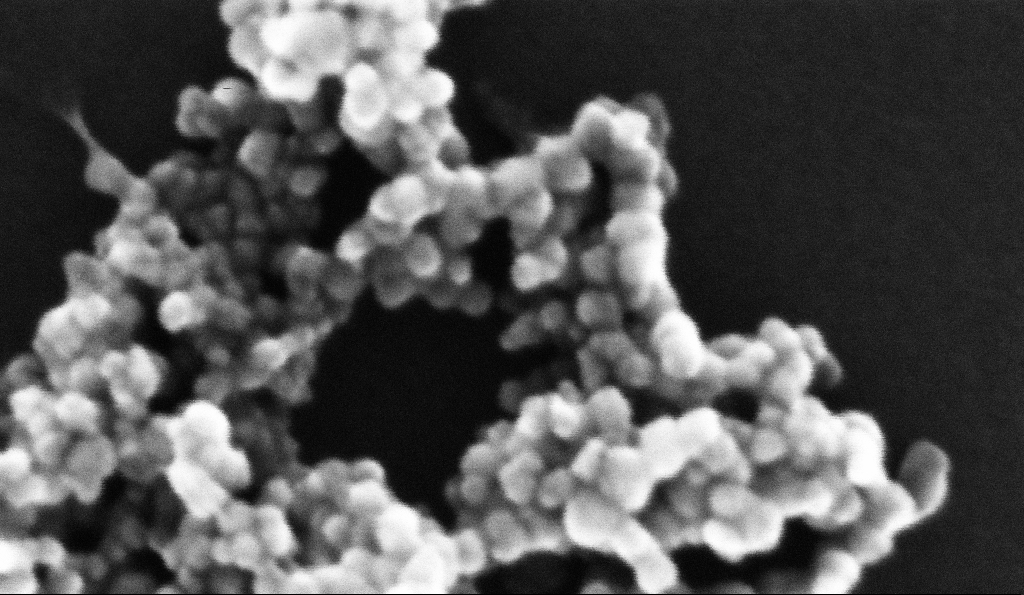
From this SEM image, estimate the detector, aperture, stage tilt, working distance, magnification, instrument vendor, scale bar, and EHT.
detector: InLens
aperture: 30 µm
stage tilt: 0°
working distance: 5.2 mm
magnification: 739.83 K X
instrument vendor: Zeiss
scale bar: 20 nm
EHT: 10 kV